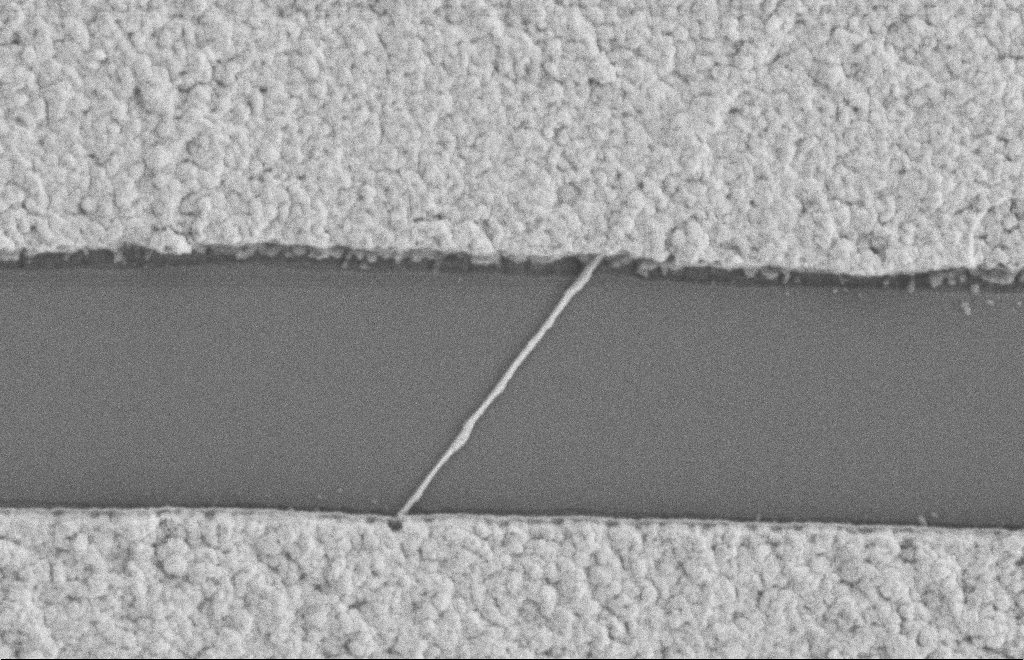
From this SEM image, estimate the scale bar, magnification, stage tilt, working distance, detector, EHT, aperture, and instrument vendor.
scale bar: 1000 nm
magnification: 40.2 K X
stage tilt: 0°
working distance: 8 mm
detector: SE2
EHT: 2 kV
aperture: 20 µm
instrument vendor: Zeiss